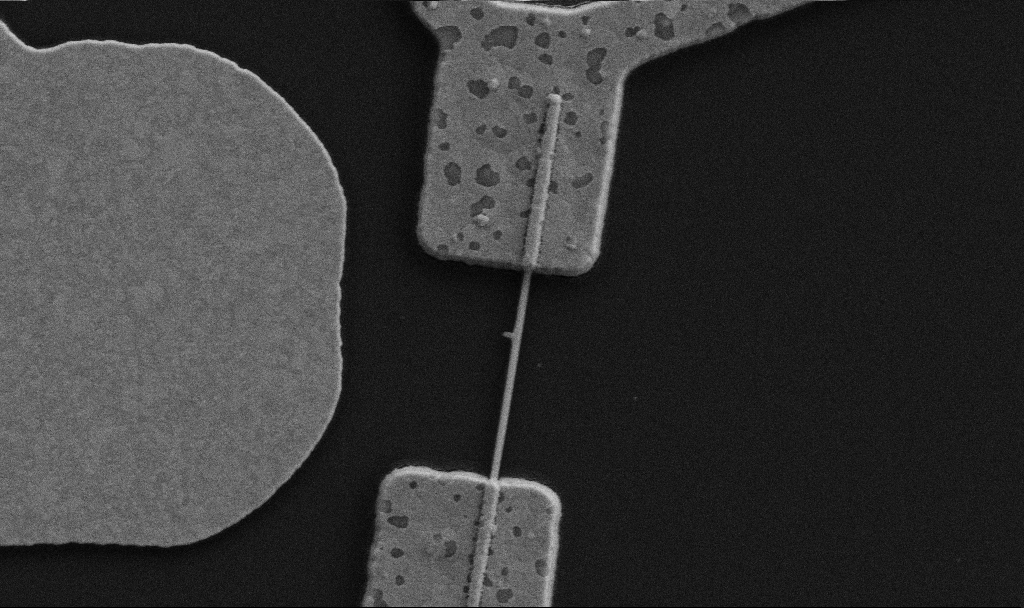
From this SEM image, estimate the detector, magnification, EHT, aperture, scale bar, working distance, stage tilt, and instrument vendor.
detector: SE2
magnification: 30 K X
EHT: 5 kV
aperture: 30 µm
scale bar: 2000 nm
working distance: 8.7 mm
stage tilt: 0°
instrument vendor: Zeiss